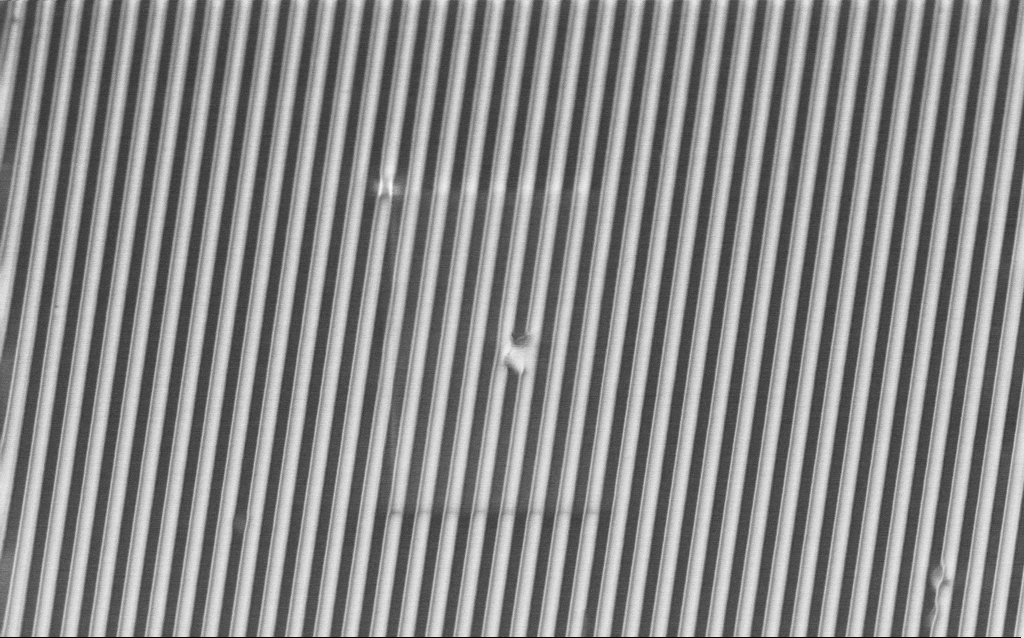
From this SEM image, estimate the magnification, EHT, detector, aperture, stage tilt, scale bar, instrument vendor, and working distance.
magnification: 20.62 K X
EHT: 2 kV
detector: InLens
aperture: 30 µm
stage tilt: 45°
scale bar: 2000 nm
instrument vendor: Zeiss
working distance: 4.1 mm